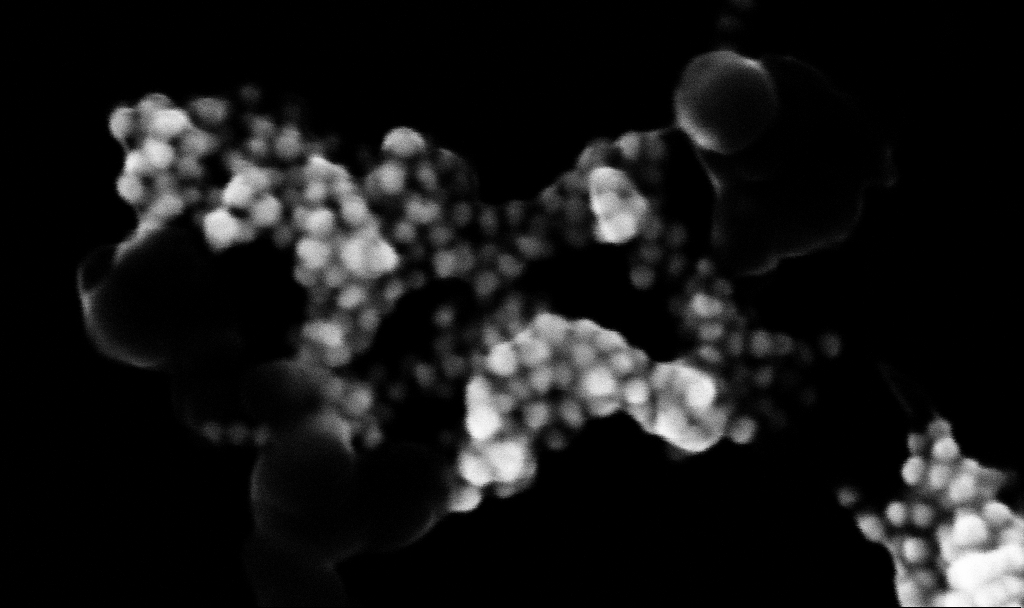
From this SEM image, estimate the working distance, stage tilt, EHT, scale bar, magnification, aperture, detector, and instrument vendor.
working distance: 3.4 mm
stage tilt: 0°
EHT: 10 kV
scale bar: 100 nm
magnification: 471.03 K X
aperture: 30 µm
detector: InLens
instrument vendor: Zeiss